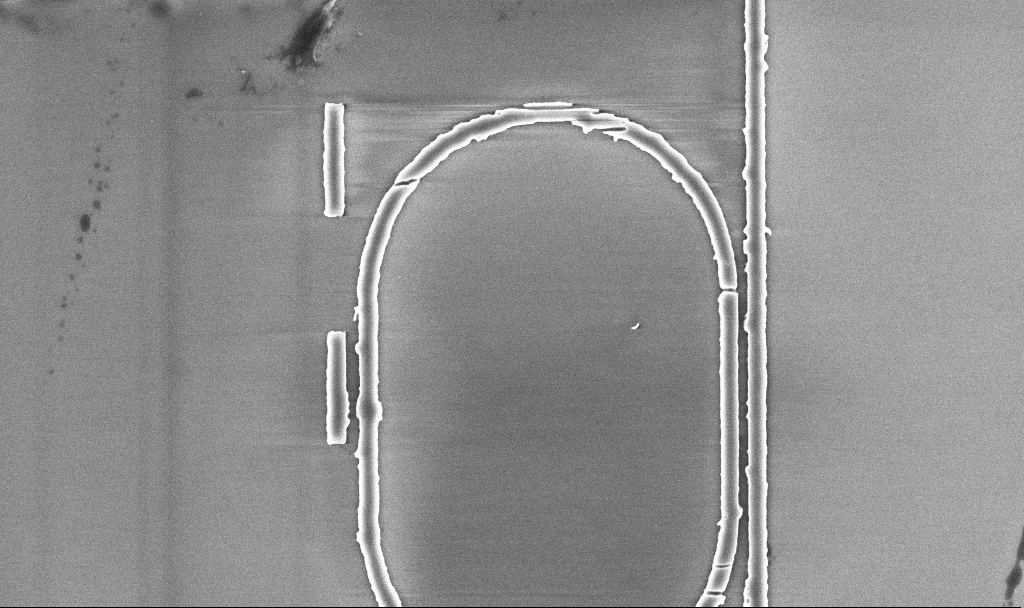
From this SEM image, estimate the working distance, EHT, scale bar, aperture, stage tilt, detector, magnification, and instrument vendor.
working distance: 5.2 mm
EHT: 5 kV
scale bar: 2000 nm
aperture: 30 µm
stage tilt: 0°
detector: InLens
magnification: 14.11 K X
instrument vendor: Zeiss